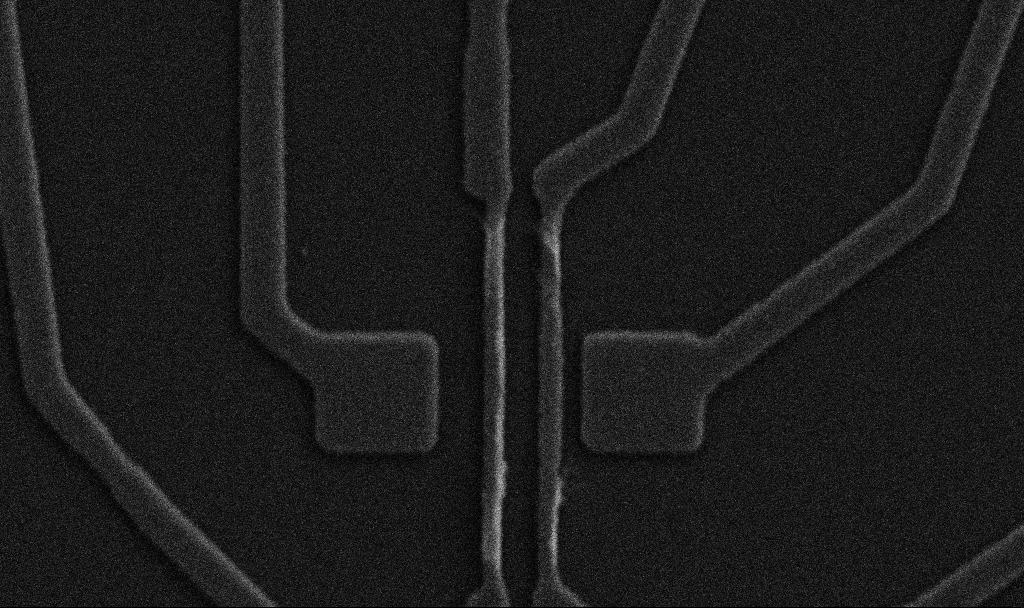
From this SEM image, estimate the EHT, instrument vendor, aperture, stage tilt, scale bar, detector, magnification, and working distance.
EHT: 5 kV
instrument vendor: Zeiss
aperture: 30 µm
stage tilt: -0°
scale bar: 1000 nm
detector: SE2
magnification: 20 K X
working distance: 10.7 mm